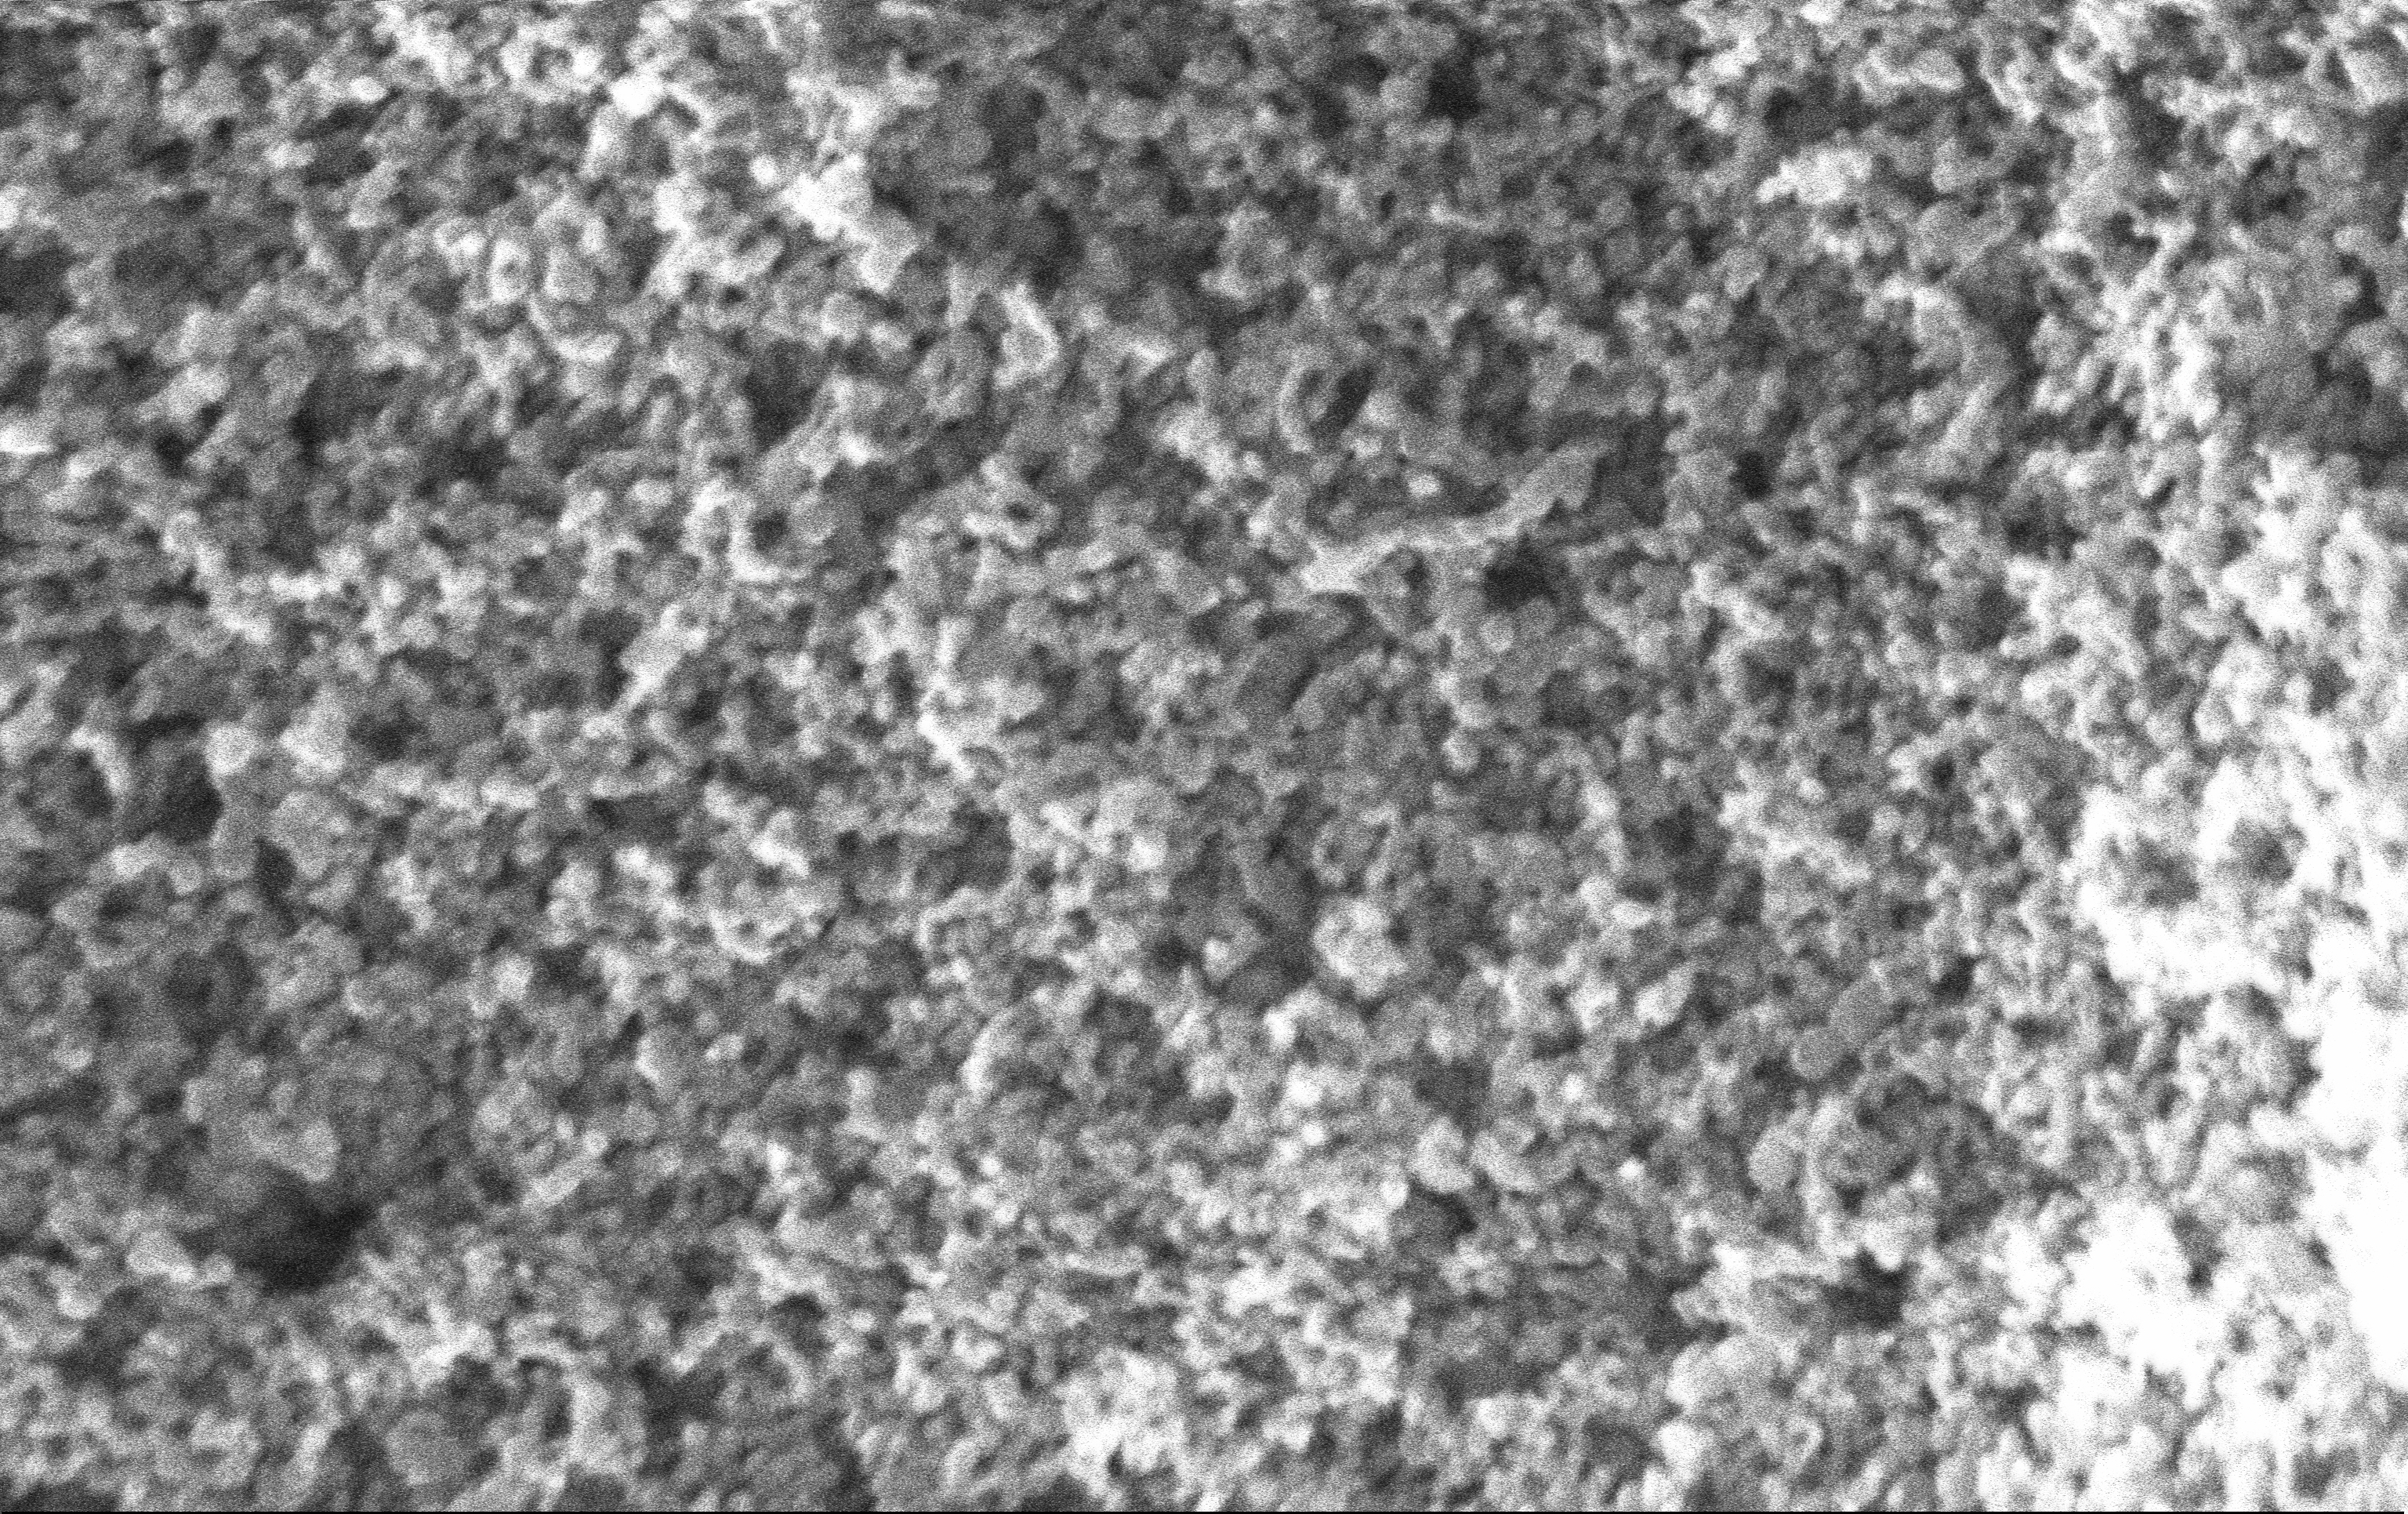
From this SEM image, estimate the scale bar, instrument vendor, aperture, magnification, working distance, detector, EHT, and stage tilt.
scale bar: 200 nm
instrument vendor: Zeiss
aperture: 30 µm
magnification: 135 K X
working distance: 2.6 mm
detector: InLens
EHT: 10 kV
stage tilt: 0°